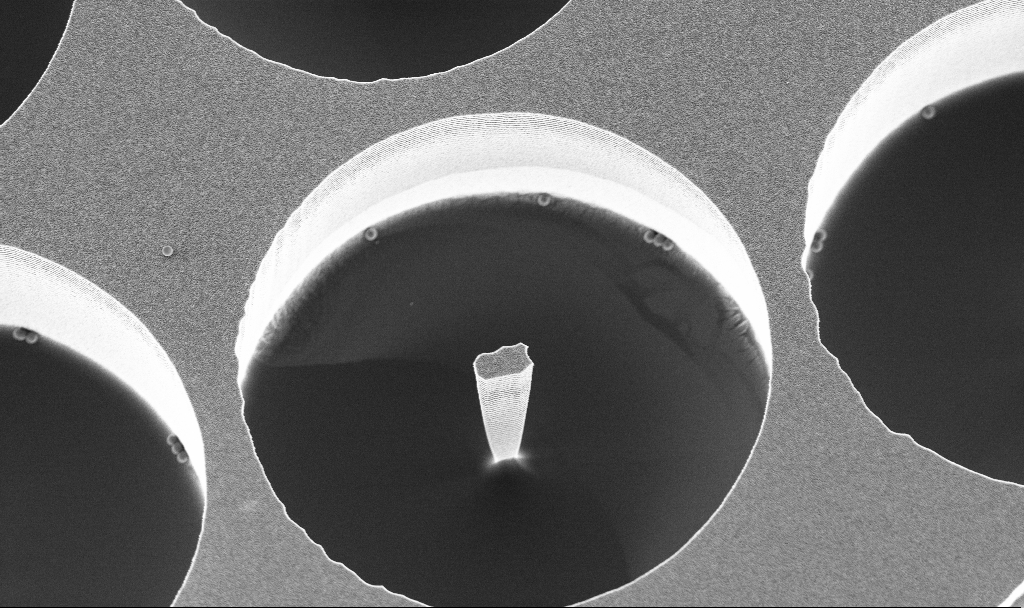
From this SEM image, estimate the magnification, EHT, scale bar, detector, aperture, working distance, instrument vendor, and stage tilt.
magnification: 6.49 K X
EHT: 3 kV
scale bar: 10000 nm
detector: InLens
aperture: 30 µm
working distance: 3.8 mm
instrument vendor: Zeiss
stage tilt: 20°